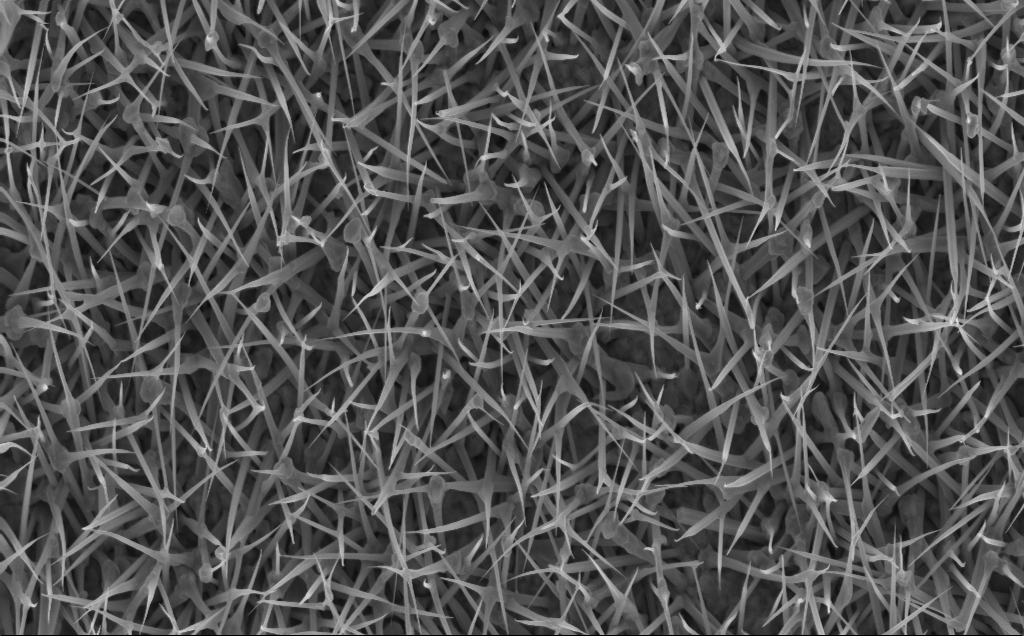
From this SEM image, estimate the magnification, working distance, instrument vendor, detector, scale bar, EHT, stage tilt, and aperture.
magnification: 20 K X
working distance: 5 mm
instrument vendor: Zeiss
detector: InLens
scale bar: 1000 nm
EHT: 5 kV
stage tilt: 0°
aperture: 30 µm